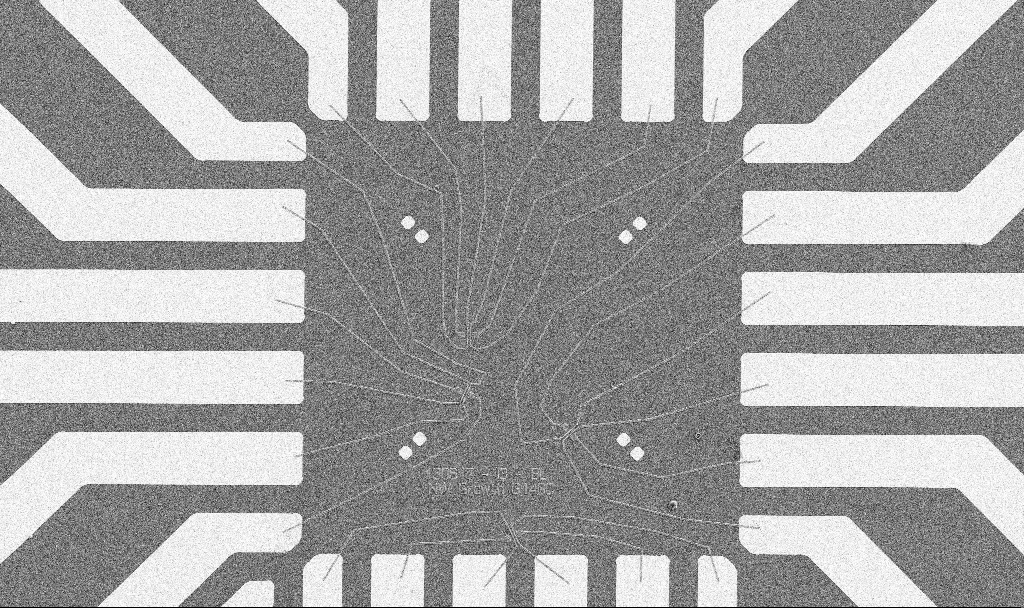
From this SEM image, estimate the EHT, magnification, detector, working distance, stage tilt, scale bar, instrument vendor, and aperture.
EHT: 5 kV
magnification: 1 K X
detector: SE2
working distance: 10.7 mm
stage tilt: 0°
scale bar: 20000 nm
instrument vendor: Zeiss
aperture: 30 µm